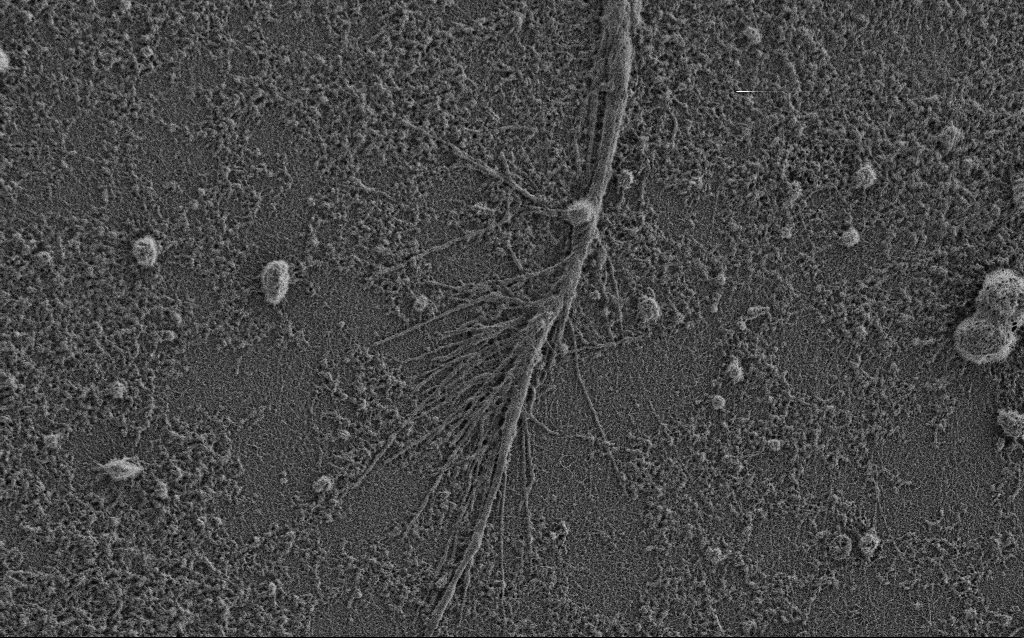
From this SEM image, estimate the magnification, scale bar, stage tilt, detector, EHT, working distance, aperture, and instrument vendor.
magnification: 7.5 K X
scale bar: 2000 nm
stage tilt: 0°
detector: SE2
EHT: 0.9 kV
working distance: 3.4 mm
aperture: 30 µm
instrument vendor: Zeiss